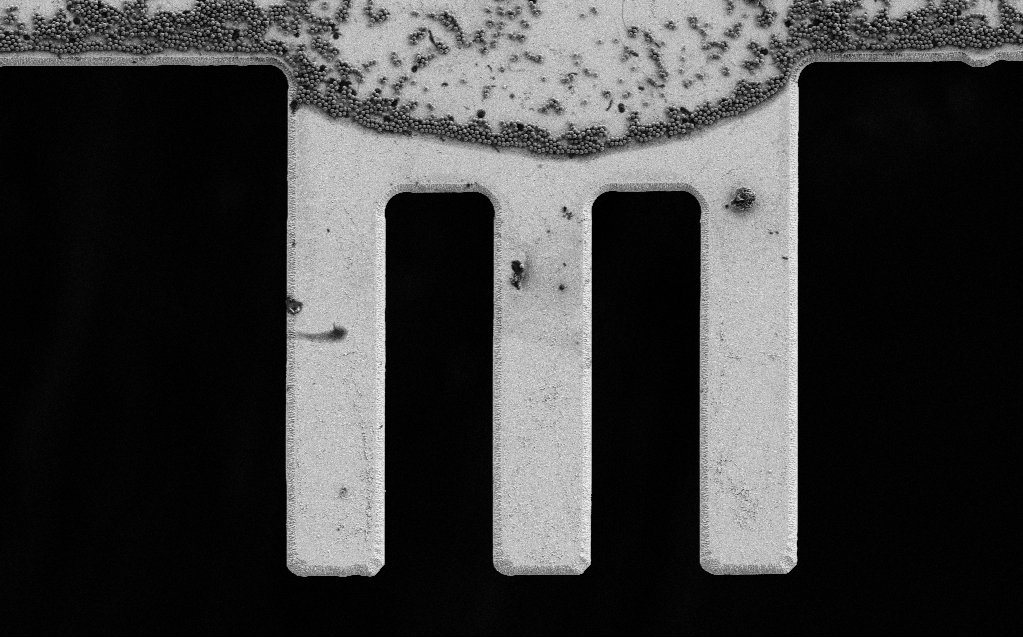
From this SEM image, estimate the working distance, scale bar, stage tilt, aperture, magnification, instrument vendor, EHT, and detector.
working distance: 7 mm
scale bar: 20000 nm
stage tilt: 0°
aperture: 30 µm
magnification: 1.9 K X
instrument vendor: Zeiss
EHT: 3 kV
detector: SE2